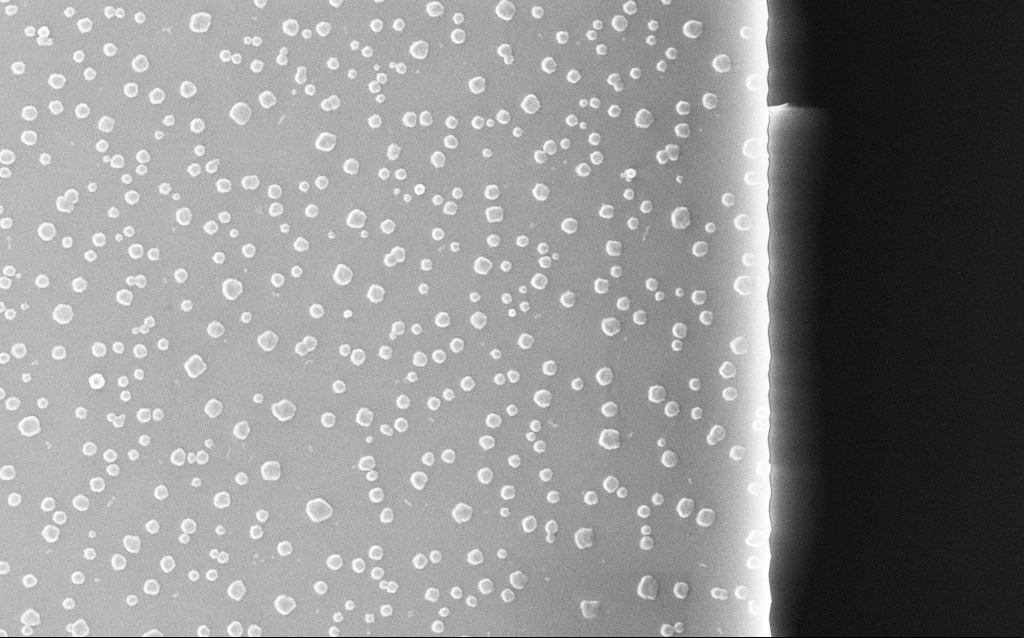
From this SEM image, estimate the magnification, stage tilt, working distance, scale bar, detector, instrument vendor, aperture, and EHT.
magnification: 10 K X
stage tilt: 0°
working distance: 1.8 mm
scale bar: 2000 nm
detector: InLens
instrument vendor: Zeiss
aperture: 30 µm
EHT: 20 kV